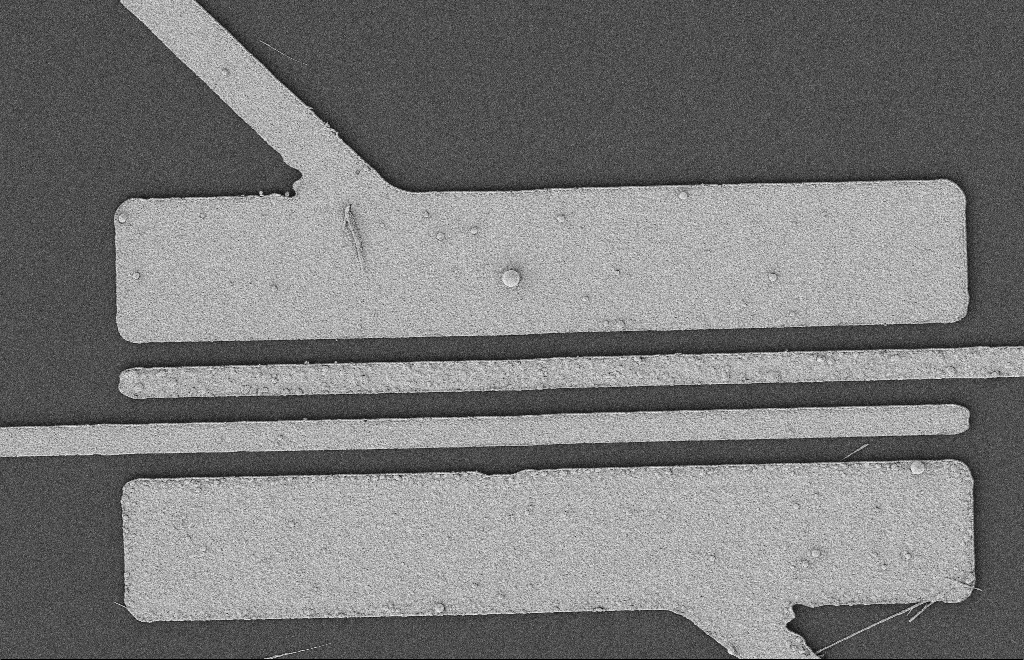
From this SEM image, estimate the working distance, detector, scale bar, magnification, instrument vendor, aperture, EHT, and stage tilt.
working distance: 12 mm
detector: SE2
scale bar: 2000 nm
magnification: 5.08 K X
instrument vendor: Zeiss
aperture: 20 µm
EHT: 2 kV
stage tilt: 0°